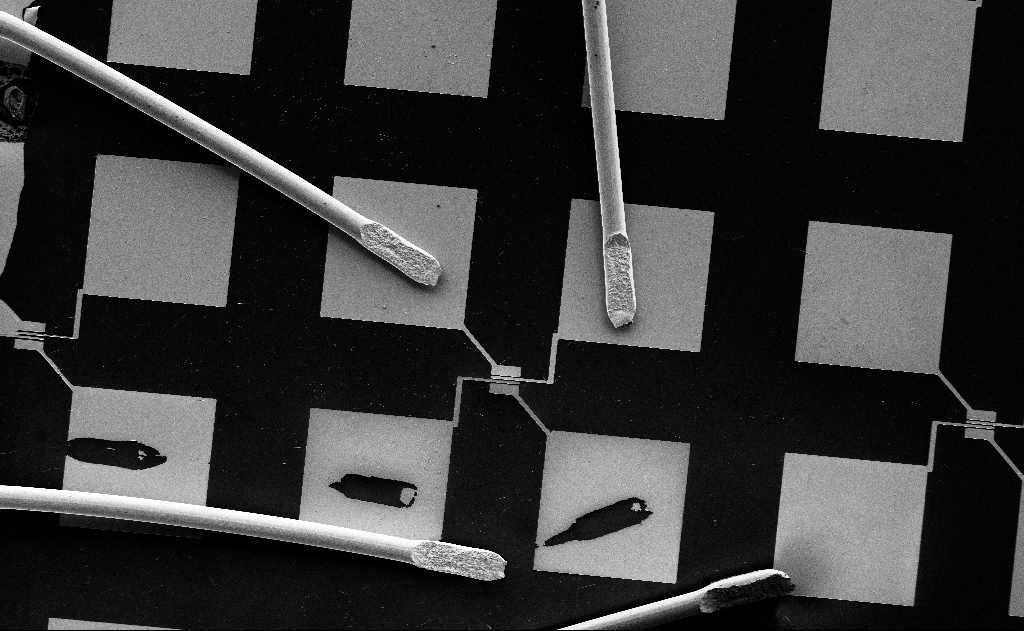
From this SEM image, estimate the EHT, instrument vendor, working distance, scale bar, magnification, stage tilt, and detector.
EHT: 5 kV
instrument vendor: Zeiss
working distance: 15 mm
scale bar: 100000 nm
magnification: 0.354 K X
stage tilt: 0°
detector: SE2